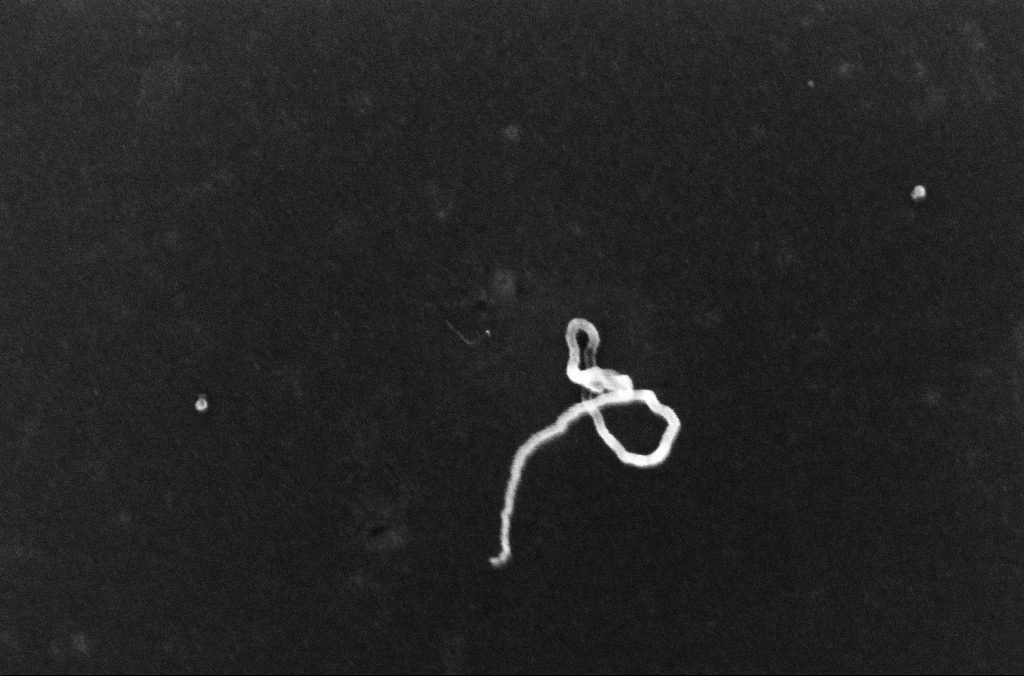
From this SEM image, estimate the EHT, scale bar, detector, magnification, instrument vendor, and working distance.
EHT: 10 kV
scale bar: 200 nm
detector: InLens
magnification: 209.1 K X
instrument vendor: Zeiss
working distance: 3.2 mm